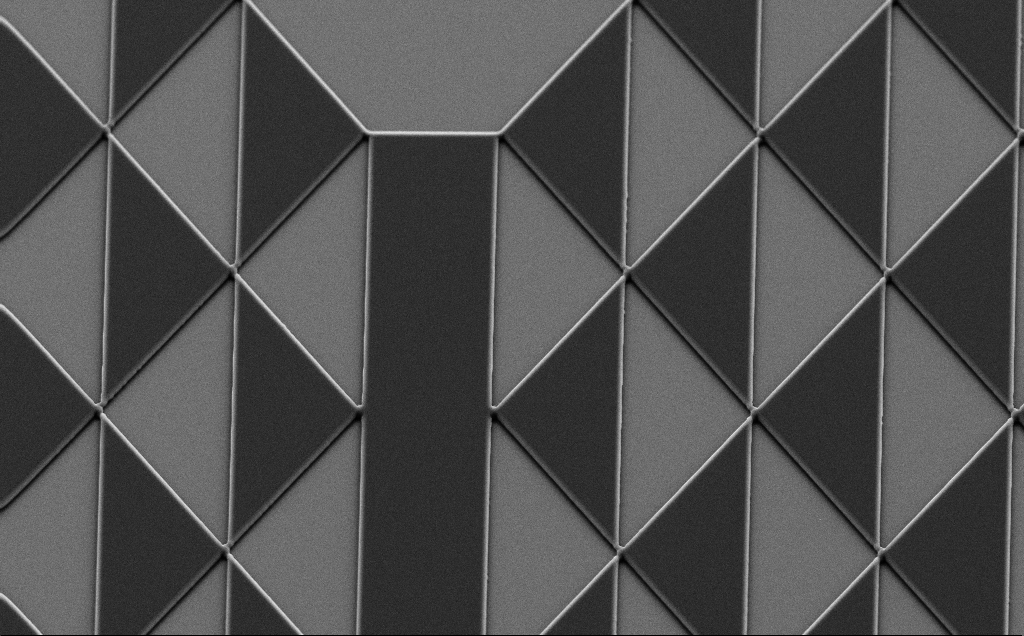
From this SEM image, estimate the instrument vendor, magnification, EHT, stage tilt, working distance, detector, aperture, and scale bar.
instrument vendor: Zeiss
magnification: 1.36 K X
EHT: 10 kV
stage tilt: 35°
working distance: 8 mm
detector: SE2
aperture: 30 µm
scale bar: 10000 nm